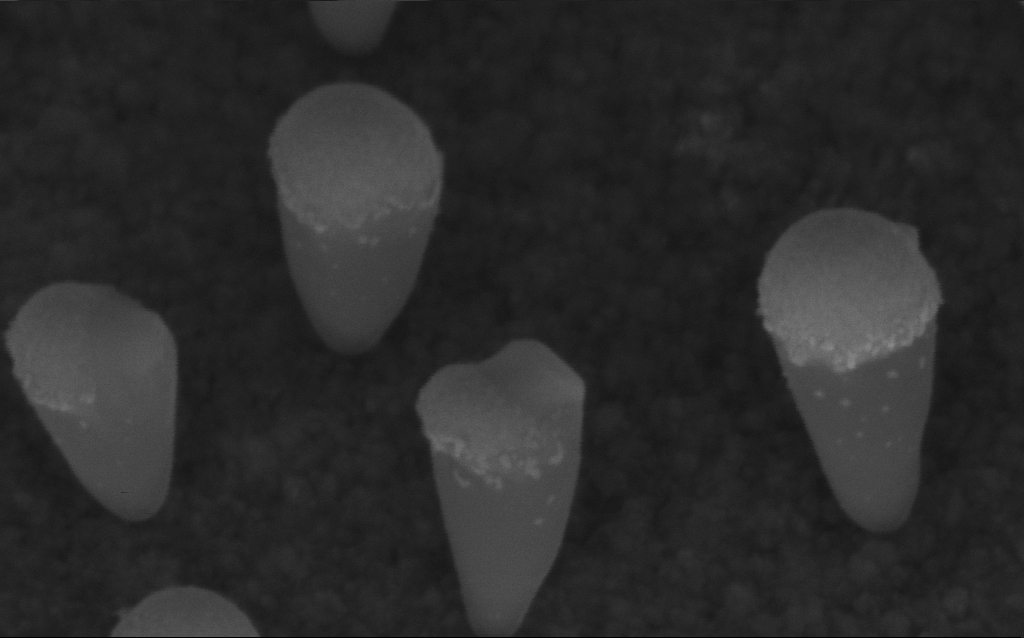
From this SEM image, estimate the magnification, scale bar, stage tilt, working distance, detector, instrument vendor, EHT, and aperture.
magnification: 378.28 K X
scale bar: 100 nm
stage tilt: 45°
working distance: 6.3 mm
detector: InLens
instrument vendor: Zeiss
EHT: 5 kV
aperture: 30 µm